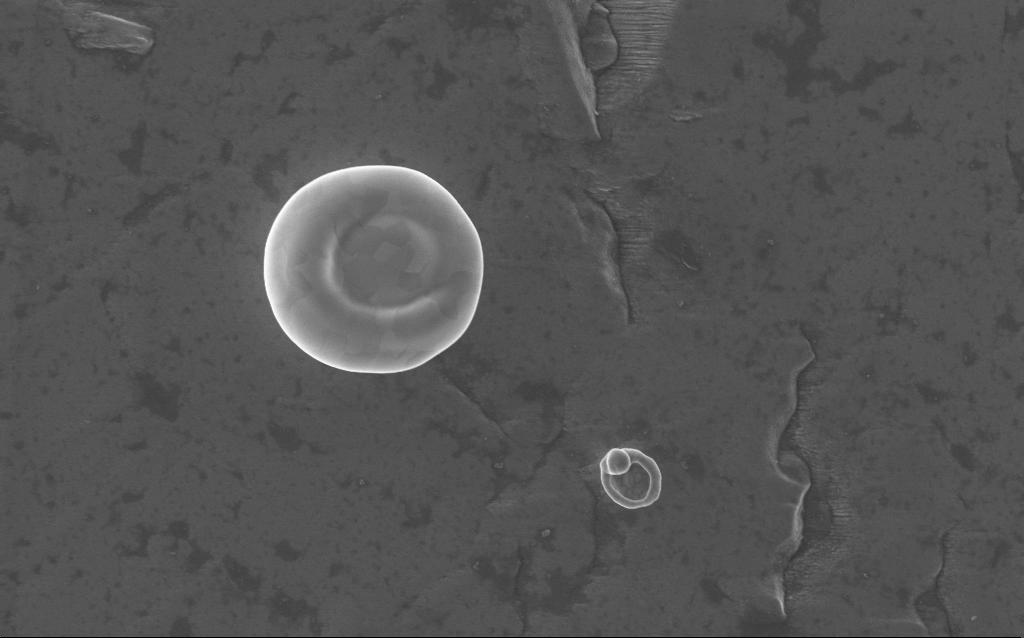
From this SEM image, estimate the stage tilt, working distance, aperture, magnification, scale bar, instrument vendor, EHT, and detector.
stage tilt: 0°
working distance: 2 mm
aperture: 30 µm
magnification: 24 K X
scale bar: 2000 nm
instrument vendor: Zeiss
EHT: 5 kV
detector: InLens